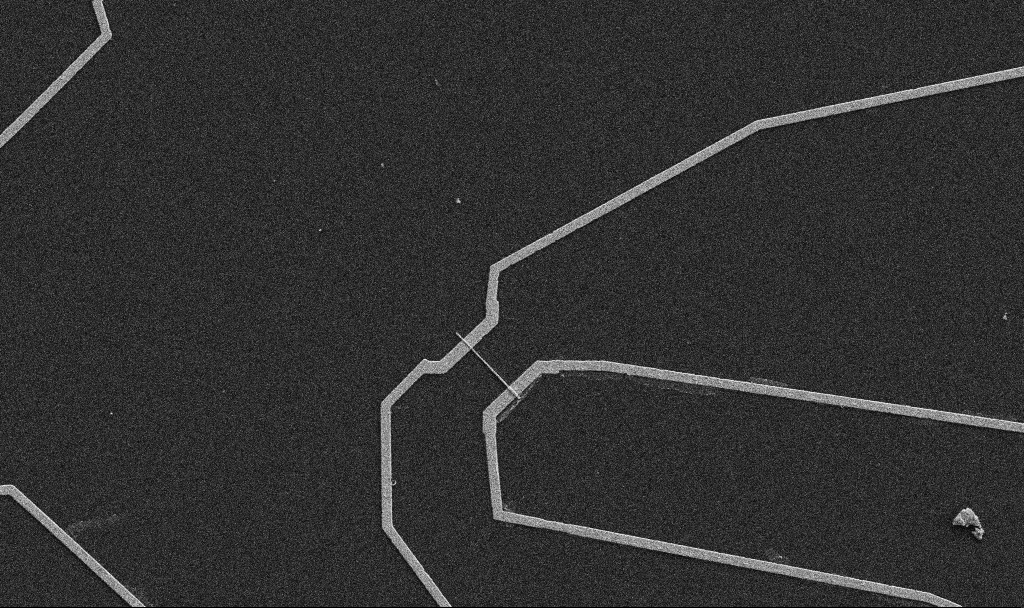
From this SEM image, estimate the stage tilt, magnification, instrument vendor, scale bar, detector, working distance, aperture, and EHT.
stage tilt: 0°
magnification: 5 K X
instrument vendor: Zeiss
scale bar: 10000 nm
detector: SE2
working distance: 10.7 mm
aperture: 30 µm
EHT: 5 kV